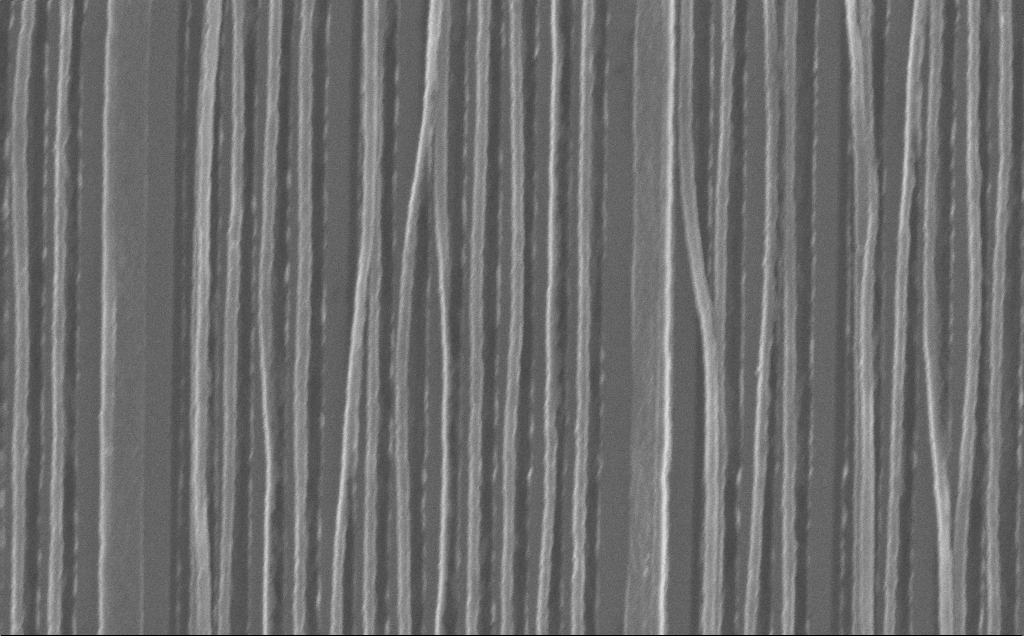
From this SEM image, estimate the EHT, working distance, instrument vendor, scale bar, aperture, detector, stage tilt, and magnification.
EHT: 10 kV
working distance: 7 mm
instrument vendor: Zeiss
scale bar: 1000 nm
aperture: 30 µm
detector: InLens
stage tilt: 0°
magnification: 64.27 K X